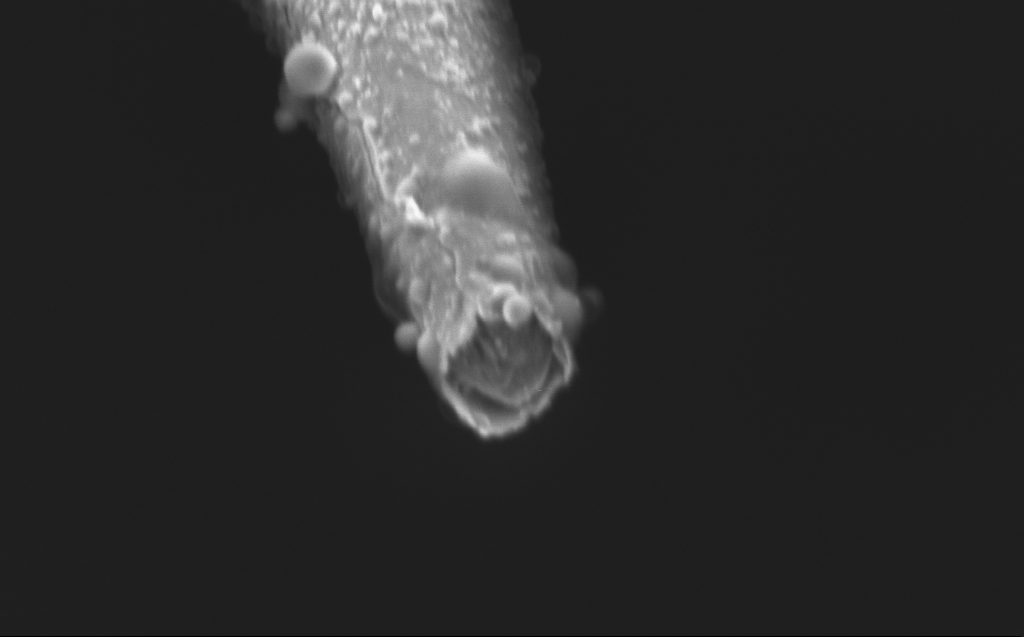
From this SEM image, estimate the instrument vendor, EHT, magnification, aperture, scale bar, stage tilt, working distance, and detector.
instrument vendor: Zeiss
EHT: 1 kV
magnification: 100 K X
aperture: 30 µm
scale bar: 200 nm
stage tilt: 45°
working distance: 4 mm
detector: InLens